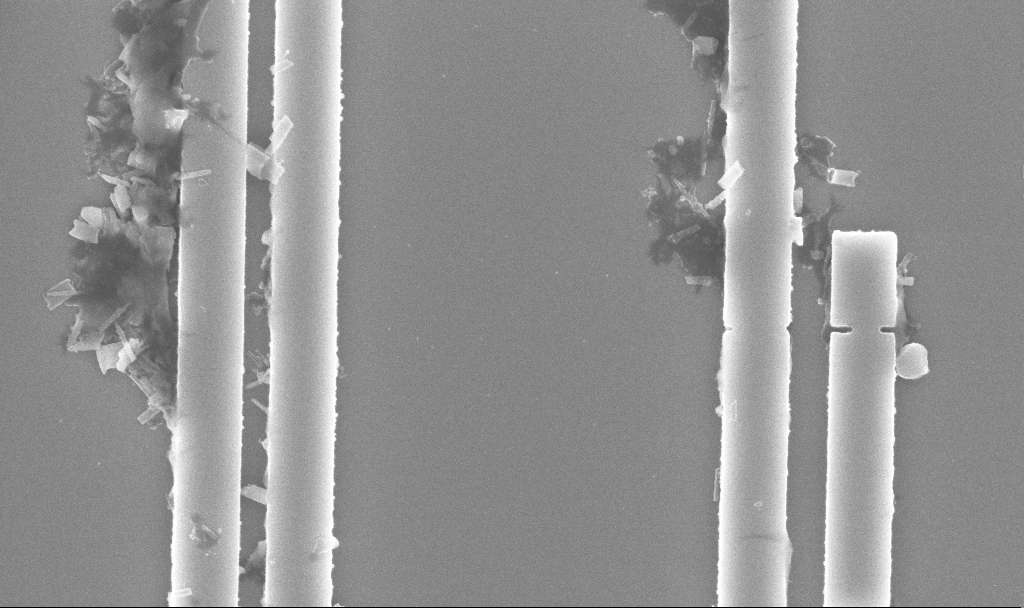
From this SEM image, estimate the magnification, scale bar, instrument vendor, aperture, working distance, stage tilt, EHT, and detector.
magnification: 47.64 K X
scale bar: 1000 nm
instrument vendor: Zeiss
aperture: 30 µm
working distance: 9.8 mm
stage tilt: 0°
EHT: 5 kV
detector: InLens